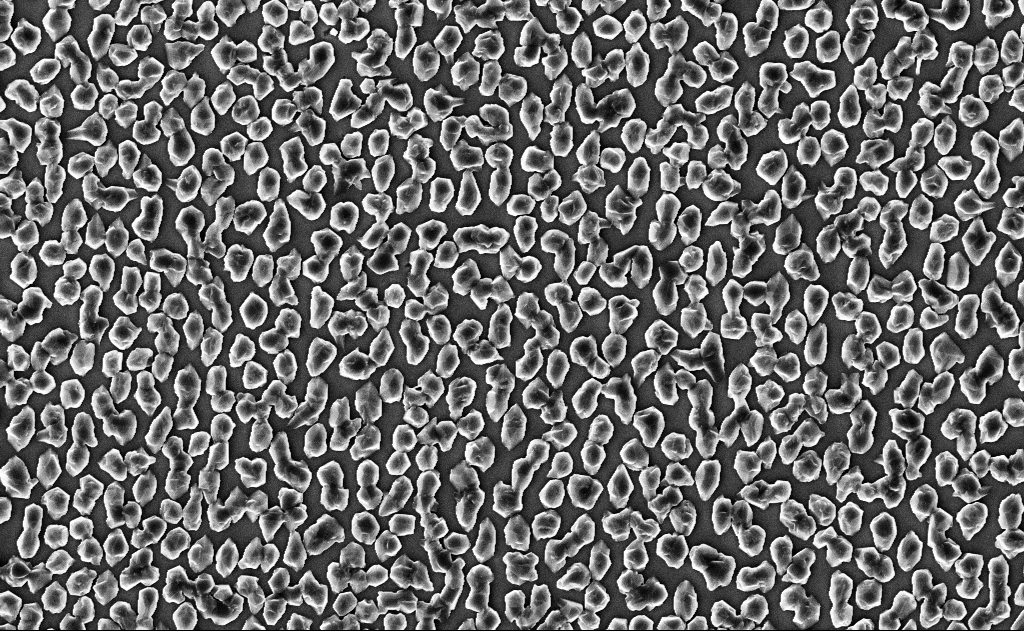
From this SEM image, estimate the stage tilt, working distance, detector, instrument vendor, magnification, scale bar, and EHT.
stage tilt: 0°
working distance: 12 mm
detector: InLens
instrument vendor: Zeiss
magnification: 10 K X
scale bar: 2000 nm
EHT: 10 kV